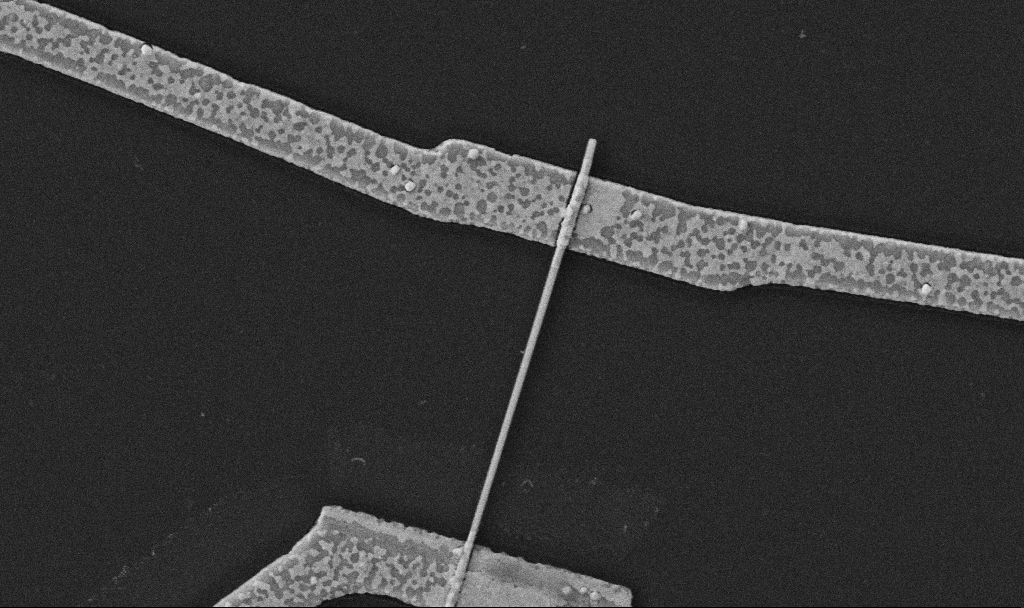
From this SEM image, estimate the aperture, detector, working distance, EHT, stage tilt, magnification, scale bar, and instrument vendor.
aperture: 30 µm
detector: SE2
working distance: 10.7 mm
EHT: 5 kV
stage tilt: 0°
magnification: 30 K X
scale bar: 1000 nm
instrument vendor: Zeiss